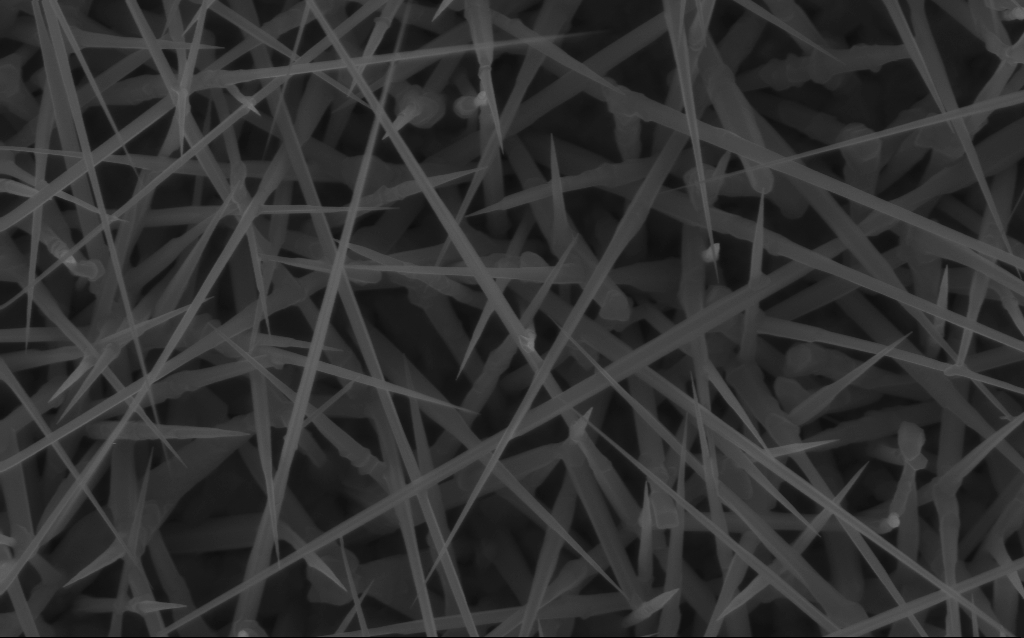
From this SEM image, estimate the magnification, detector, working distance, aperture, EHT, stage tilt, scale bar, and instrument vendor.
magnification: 40 K X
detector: InLens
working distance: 7 mm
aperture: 30 µm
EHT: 10 kV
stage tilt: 0°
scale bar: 1000 nm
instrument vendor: Zeiss